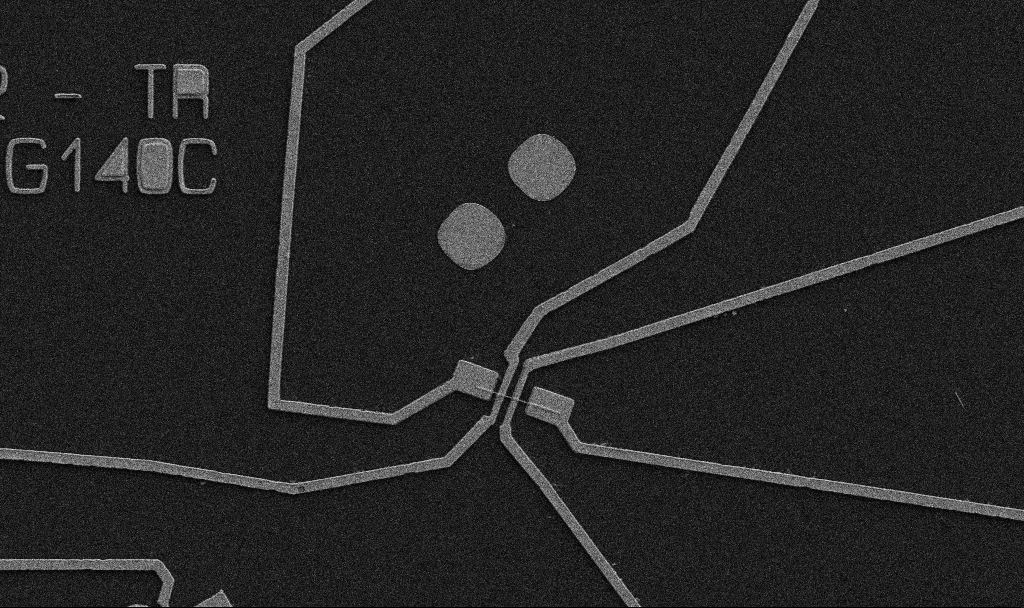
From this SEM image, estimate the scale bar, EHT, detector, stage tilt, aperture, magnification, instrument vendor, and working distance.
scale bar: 10000 nm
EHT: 5 kV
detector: SE2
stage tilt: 0°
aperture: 30 µm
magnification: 5 K X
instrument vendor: Zeiss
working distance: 10.7 mm